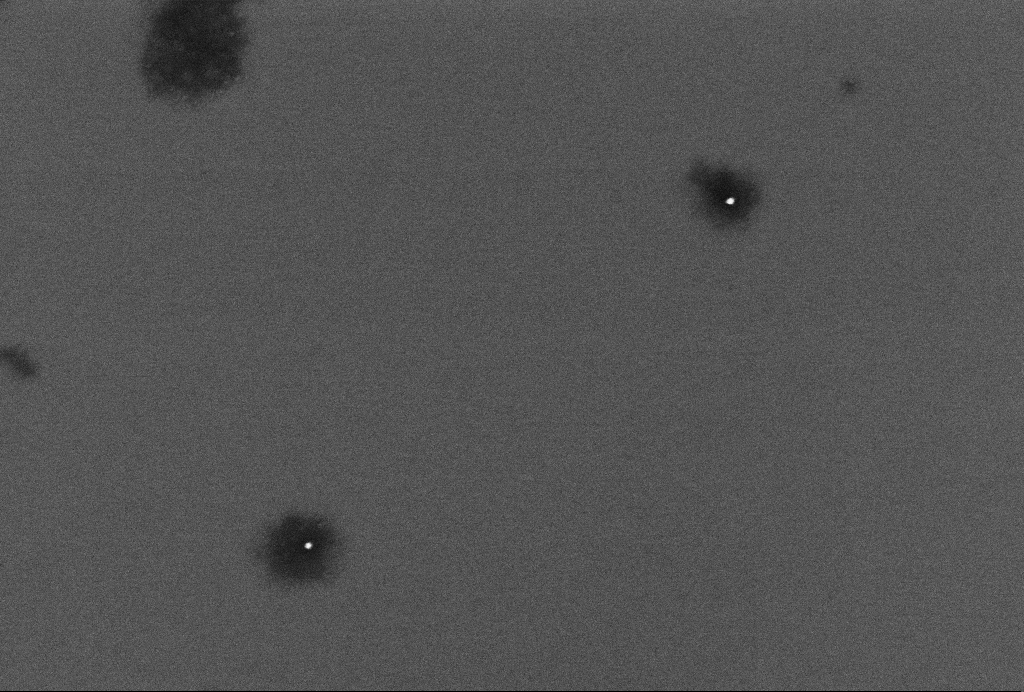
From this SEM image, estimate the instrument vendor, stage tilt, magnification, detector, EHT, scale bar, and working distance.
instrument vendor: Zeiss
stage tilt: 0°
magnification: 86.19 K X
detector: InLens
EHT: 2 kV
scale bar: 200 nm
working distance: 3.3 mm